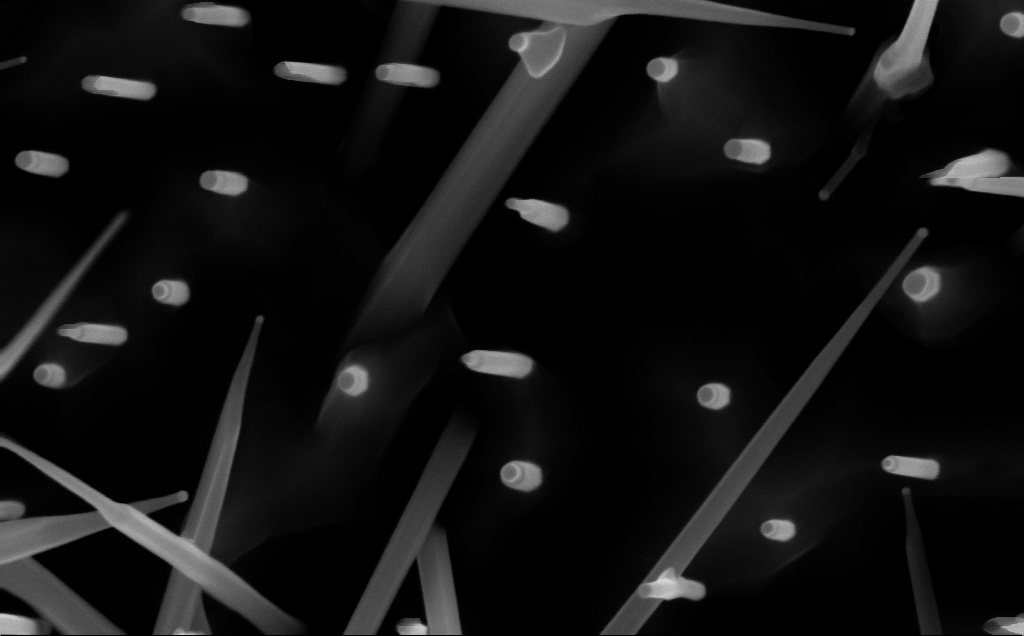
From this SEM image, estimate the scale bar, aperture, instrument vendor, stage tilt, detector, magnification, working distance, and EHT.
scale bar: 200 nm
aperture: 30 µm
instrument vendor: Zeiss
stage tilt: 0°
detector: InLens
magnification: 158.89 K X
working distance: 5 mm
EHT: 10 kV